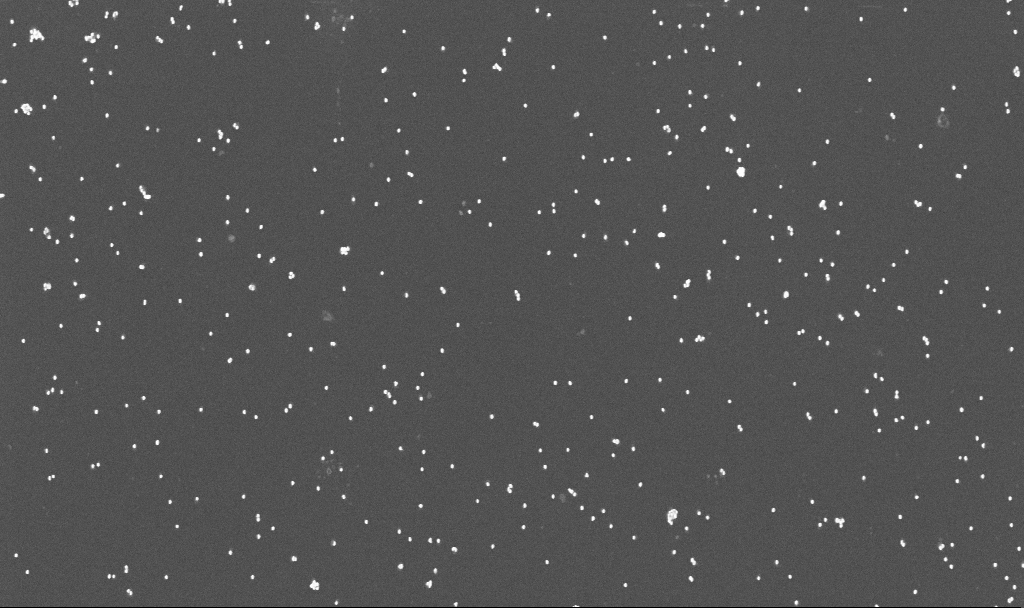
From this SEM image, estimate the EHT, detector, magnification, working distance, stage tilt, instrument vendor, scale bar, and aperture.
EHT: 10 kV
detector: InLens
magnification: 50 K X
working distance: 3.3 mm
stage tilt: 0°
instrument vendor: Zeiss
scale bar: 1000 nm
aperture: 30 µm